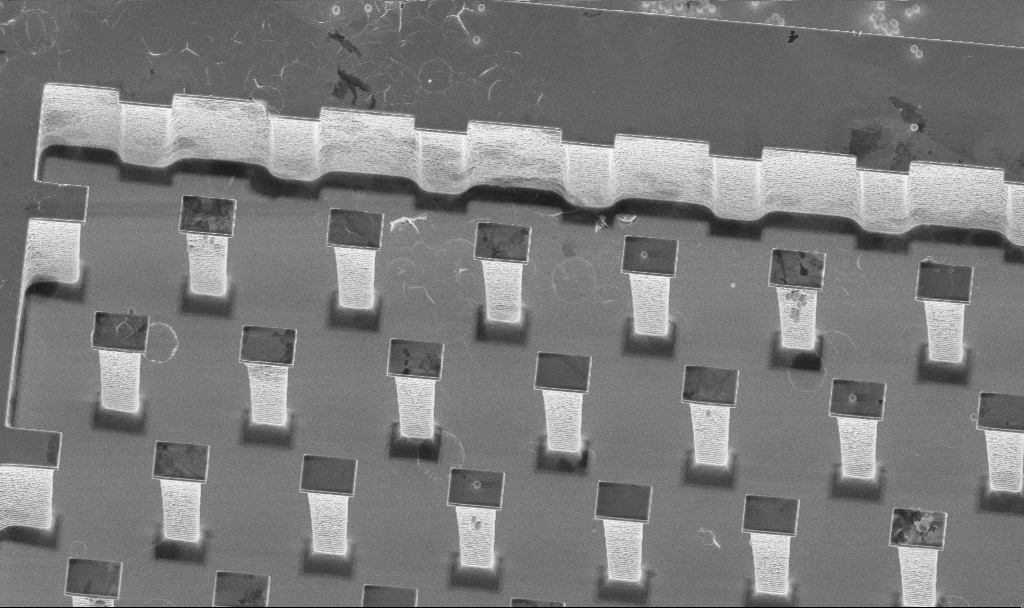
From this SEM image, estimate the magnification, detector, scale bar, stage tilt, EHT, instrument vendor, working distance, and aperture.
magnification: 4.52 K X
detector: InLens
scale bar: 10000 nm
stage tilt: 20°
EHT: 5 kV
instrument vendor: Zeiss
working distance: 5.8 mm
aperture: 30 µm